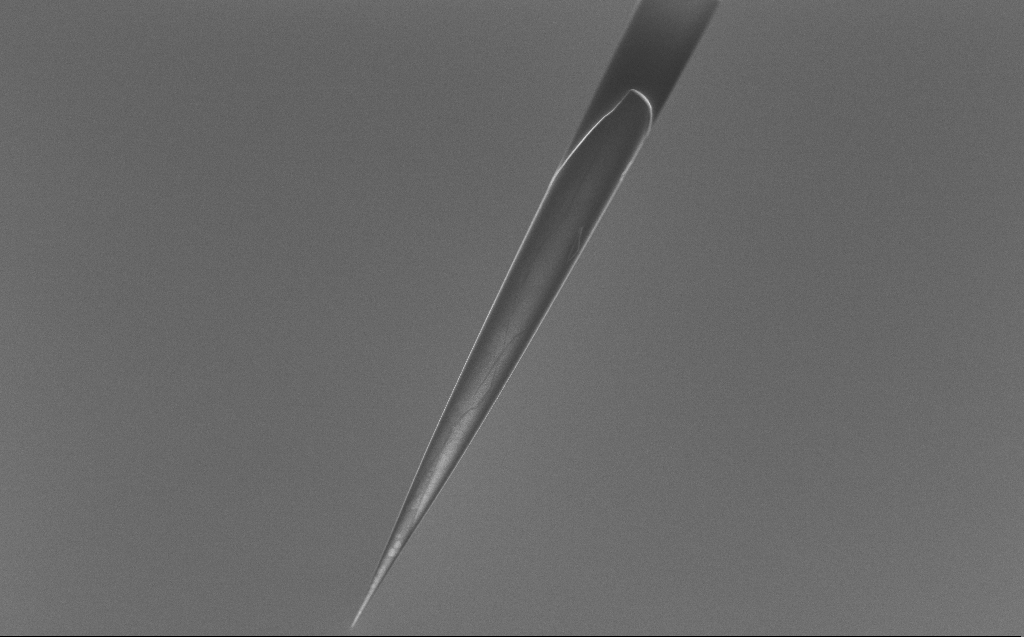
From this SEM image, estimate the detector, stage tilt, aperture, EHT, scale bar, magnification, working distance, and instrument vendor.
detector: InLens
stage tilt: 45°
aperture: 30 µm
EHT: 5 kV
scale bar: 20000 nm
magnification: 1 K X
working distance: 6 mm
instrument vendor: Zeiss